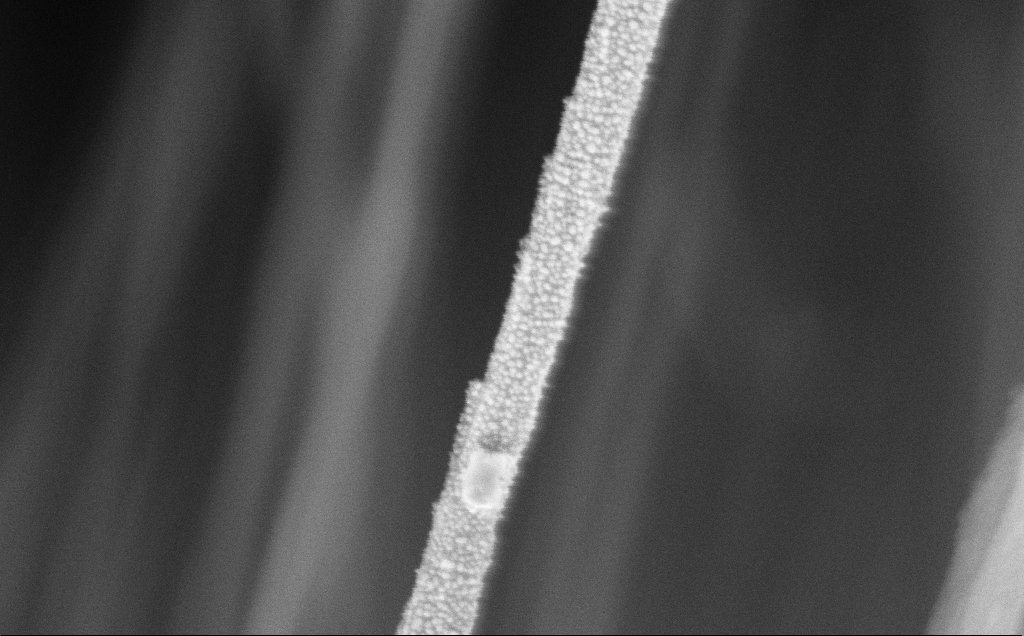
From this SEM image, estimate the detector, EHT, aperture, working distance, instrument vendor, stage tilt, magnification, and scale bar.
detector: InLens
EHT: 5 kV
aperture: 30 µm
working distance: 11 mm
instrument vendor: Zeiss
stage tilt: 0°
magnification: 163.96 K X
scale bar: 200 nm